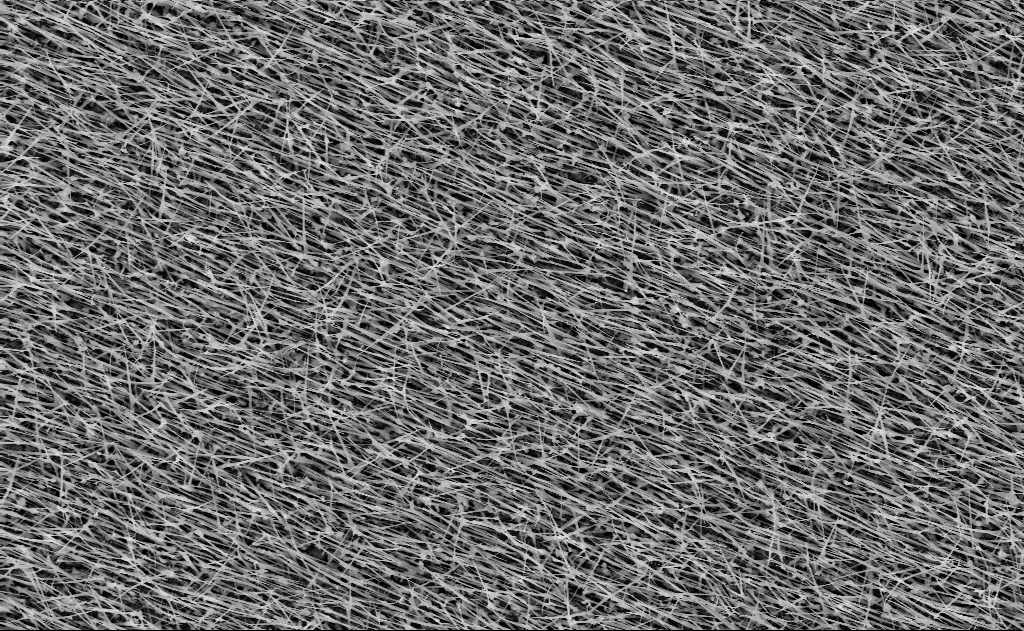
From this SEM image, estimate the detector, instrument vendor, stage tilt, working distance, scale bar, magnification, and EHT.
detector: InLens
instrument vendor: Zeiss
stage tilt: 0°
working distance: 15 mm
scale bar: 2000 nm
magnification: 10 K X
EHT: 10 kV